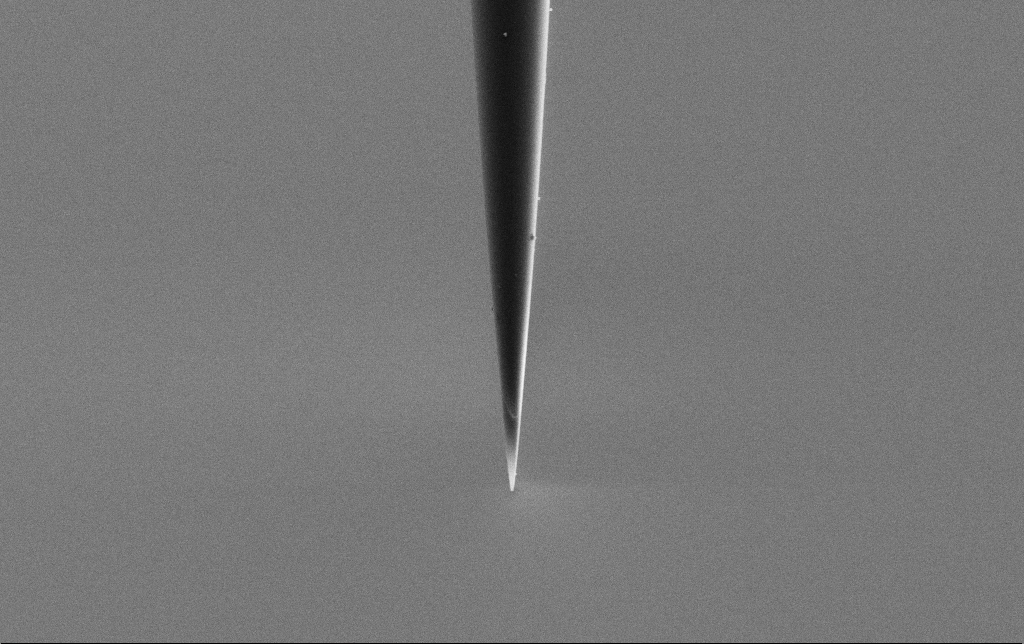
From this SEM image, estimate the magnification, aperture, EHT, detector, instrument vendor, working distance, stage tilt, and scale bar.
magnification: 15 K X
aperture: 30 µm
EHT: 2 kV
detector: SE2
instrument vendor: Zeiss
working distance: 6.6 mm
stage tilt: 0°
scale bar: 1000 nm